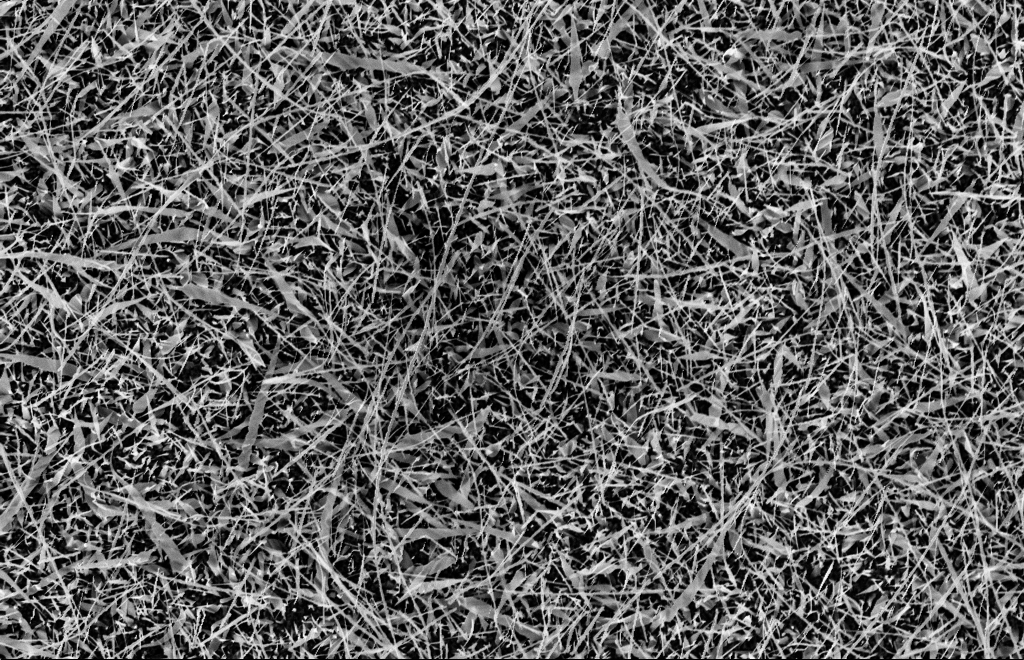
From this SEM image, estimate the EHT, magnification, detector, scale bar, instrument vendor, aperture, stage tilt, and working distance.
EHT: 10 kV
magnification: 5 K X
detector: InLens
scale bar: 2000 nm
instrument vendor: Zeiss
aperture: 30 µm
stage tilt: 0°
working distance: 15 mm